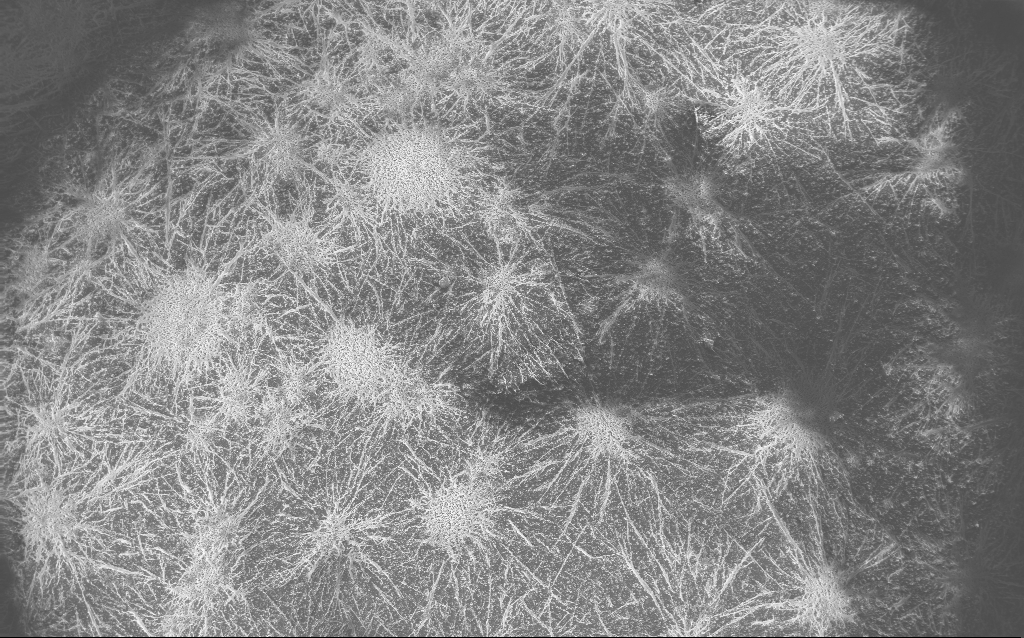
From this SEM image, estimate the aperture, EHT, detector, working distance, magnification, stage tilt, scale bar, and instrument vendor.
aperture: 30 µm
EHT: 10 kV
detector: InLens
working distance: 2.8 mm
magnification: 0.122 K X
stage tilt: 0°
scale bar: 100000 nm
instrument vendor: Zeiss